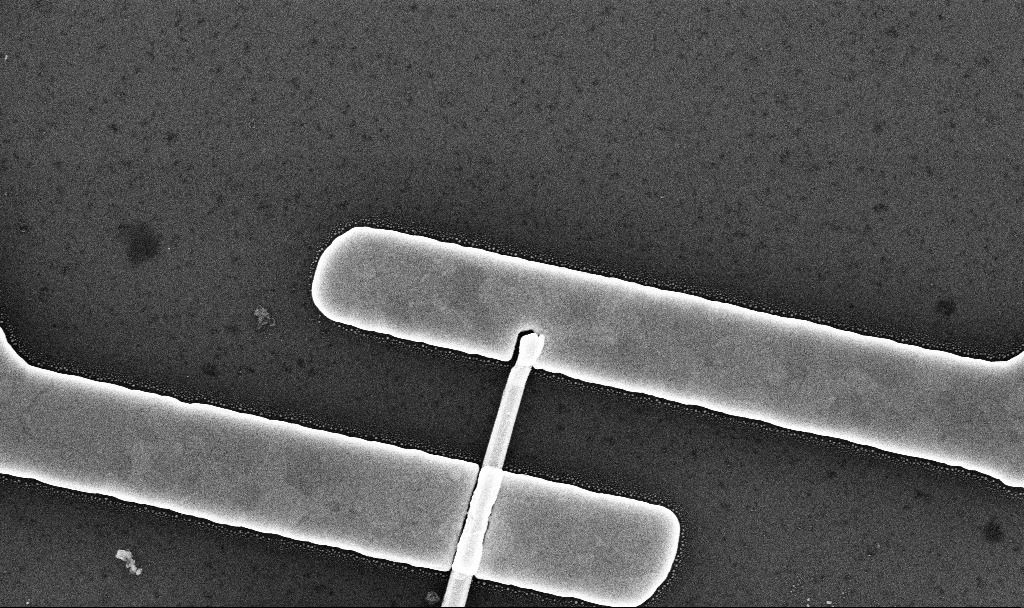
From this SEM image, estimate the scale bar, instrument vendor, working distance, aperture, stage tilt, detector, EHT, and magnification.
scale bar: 1000 nm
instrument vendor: Zeiss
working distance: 7.8 mm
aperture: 30 µm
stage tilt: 0°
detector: InLens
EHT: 10 kV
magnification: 54.57 K X